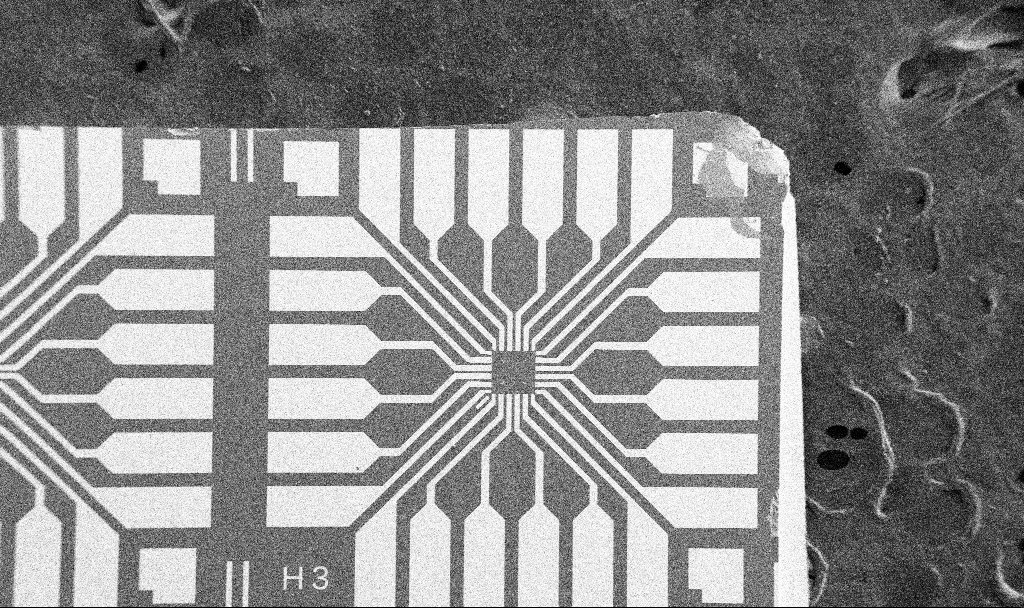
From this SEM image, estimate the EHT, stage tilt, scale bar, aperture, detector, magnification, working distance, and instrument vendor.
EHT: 5 kV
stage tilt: -0°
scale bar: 200000 nm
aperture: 30 µm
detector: SE2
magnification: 0.1 K X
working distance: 10.7 mm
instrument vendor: Zeiss